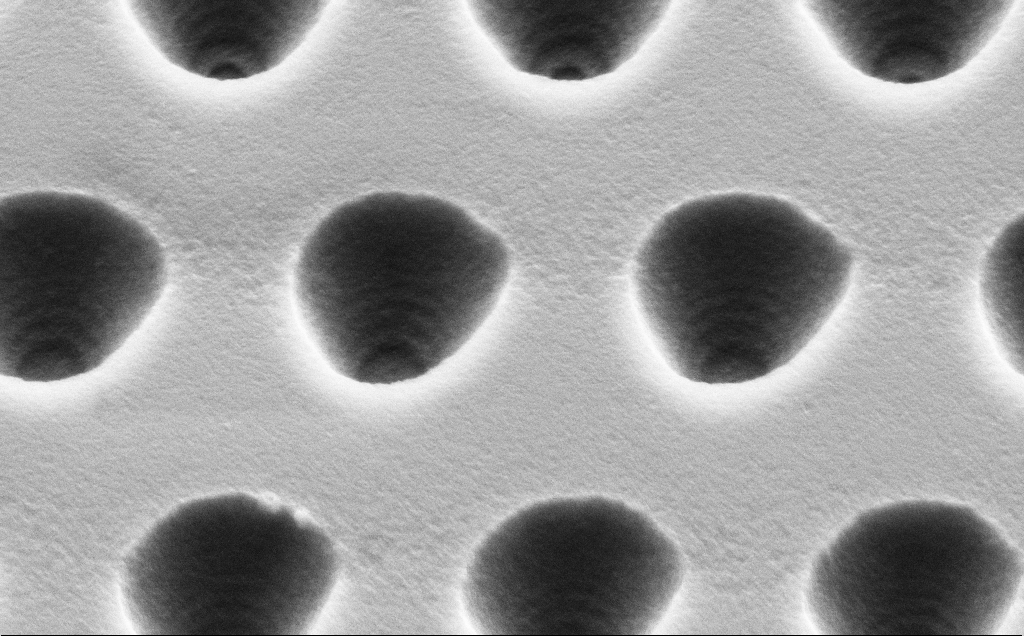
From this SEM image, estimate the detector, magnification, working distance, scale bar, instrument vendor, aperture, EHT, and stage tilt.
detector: SE2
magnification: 30.99 K X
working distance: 10 mm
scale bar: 2000 nm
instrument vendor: Zeiss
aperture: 30 µm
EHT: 5 kV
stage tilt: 45°